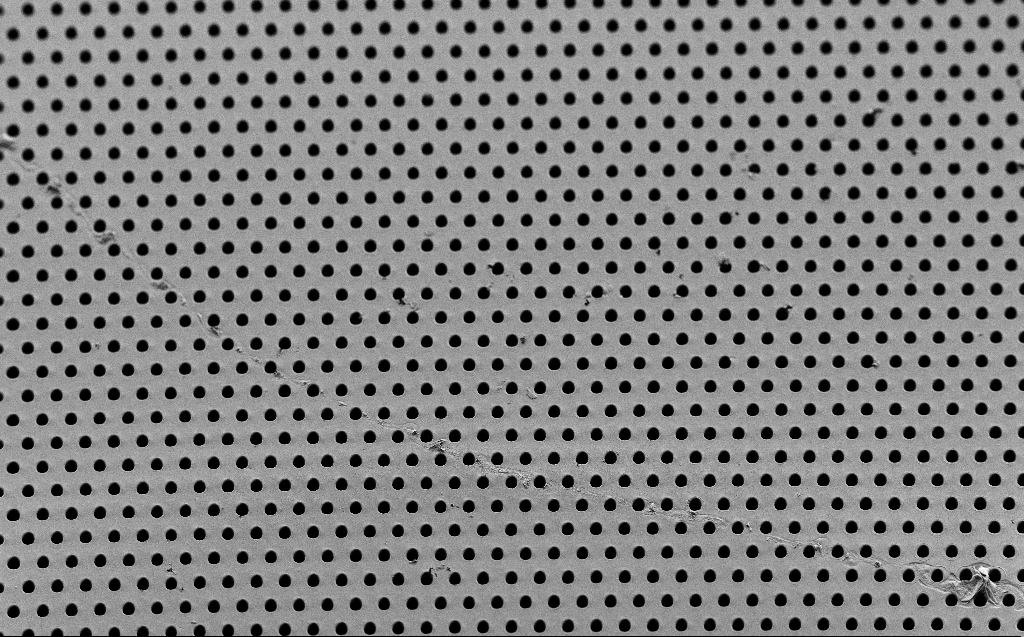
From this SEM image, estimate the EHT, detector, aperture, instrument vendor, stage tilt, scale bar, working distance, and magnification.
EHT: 2 kV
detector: SE2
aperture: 30 µm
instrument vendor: Zeiss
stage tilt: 45°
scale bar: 100000 nm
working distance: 6 mm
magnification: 0.6 K X